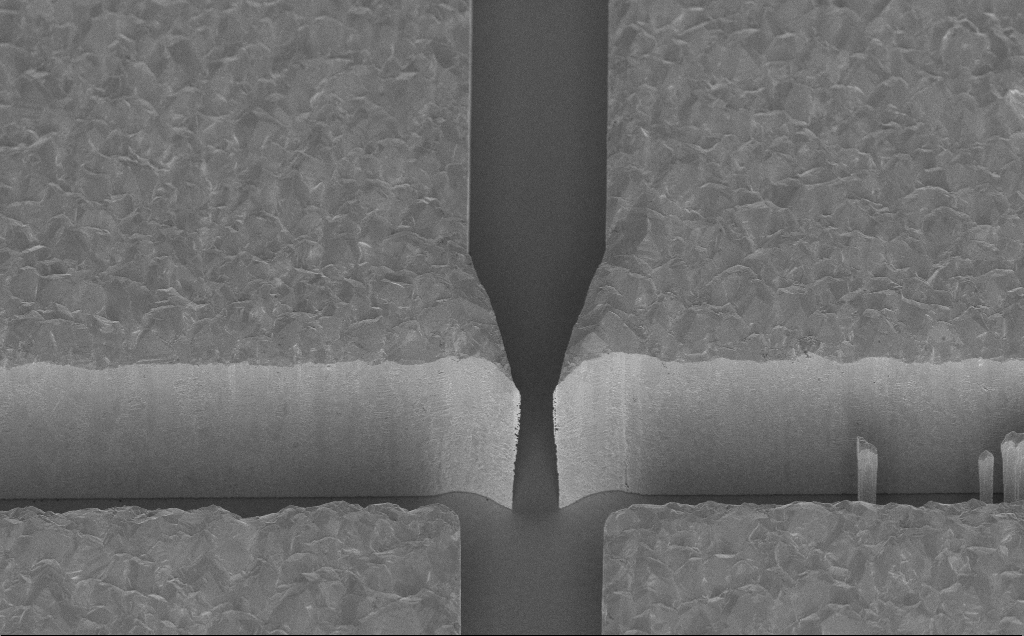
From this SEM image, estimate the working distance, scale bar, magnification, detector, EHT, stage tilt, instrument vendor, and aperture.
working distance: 10 mm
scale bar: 10000 nm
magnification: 5.12 K X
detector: InLens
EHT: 5 kV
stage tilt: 45°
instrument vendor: Zeiss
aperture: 30 µm